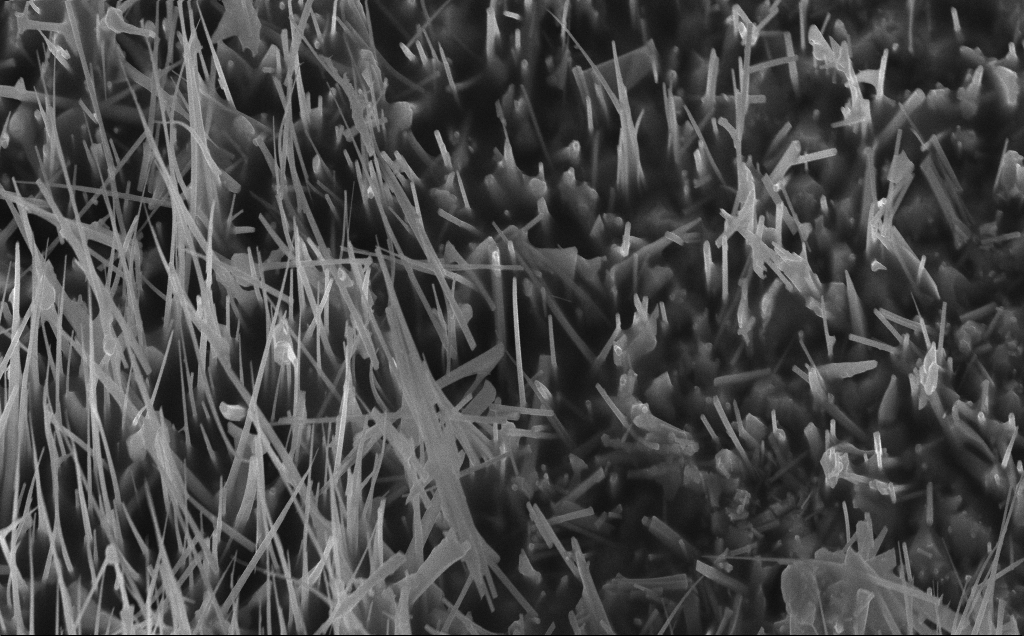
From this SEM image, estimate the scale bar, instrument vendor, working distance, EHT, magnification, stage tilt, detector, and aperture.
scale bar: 2000 nm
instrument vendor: Zeiss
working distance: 7 mm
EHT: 10 kV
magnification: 22.04 K X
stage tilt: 30°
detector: InLens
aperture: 30 µm